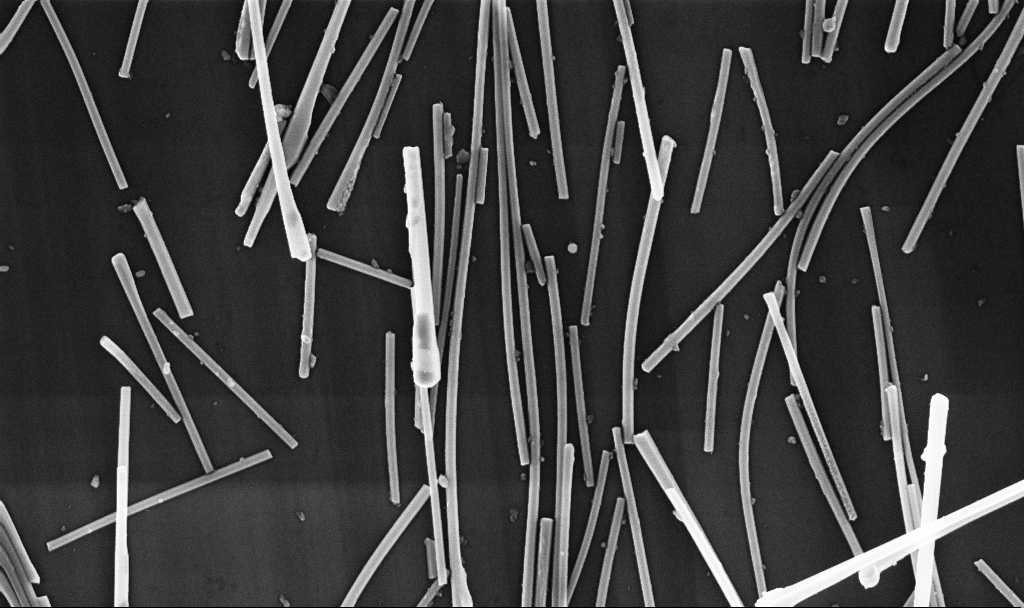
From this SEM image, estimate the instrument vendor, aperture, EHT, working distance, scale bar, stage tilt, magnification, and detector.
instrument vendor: Zeiss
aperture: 30 µm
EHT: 10 kV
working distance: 6.7 mm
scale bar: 1000 nm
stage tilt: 0°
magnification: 33.33 K X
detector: InLens